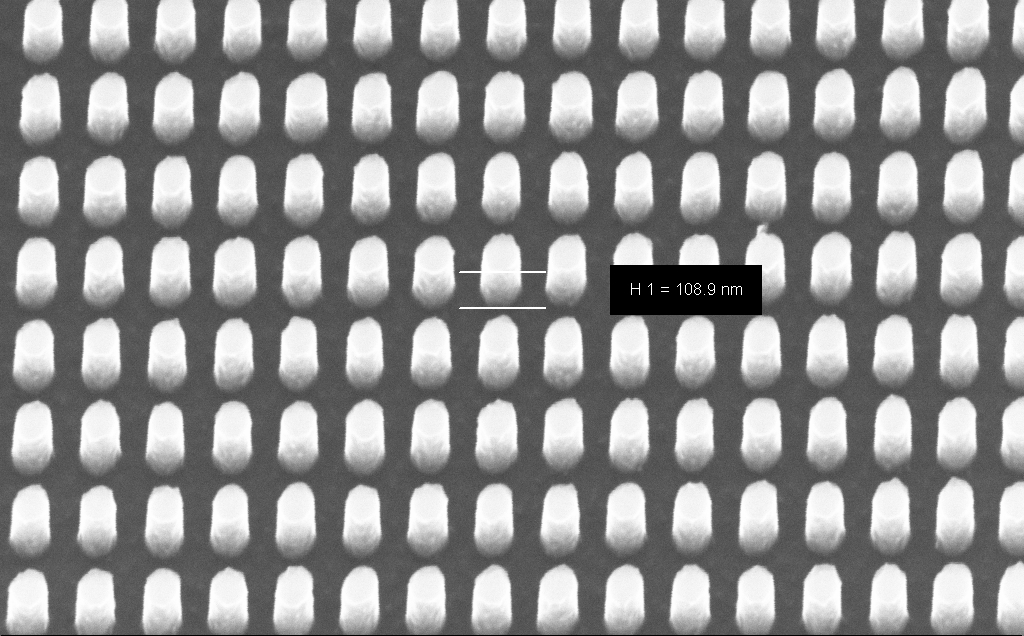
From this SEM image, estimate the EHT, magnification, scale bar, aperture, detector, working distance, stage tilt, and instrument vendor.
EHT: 10 kV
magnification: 121.38 K X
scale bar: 200 nm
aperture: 30 µm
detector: InLens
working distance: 6 mm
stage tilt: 30°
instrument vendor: Zeiss